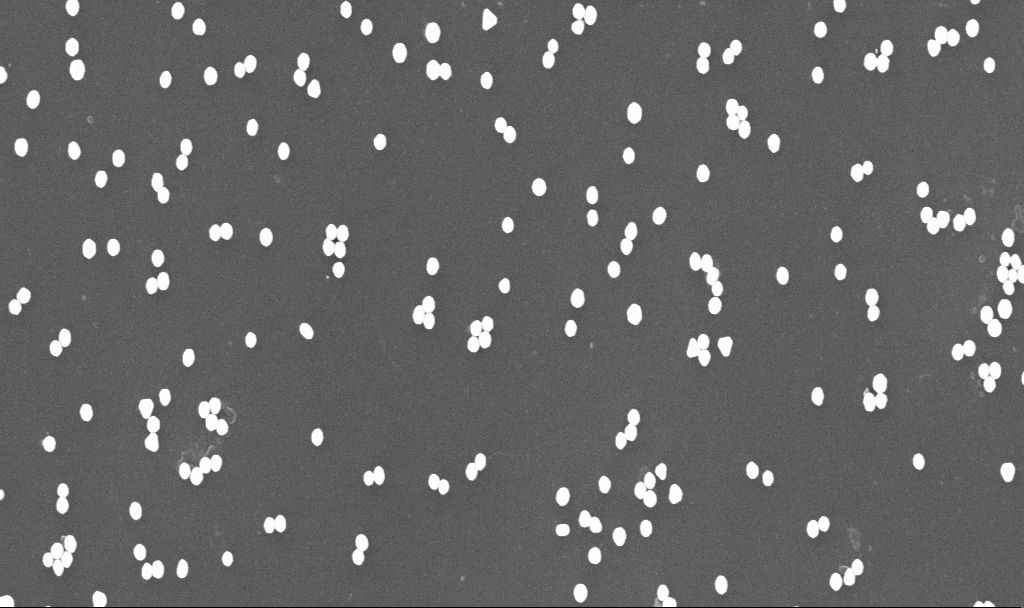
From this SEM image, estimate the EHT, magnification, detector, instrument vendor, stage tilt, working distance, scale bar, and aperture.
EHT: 10 kV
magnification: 70 K X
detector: InLens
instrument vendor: Zeiss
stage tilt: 0°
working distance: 3.4 mm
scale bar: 1000 nm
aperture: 30 µm